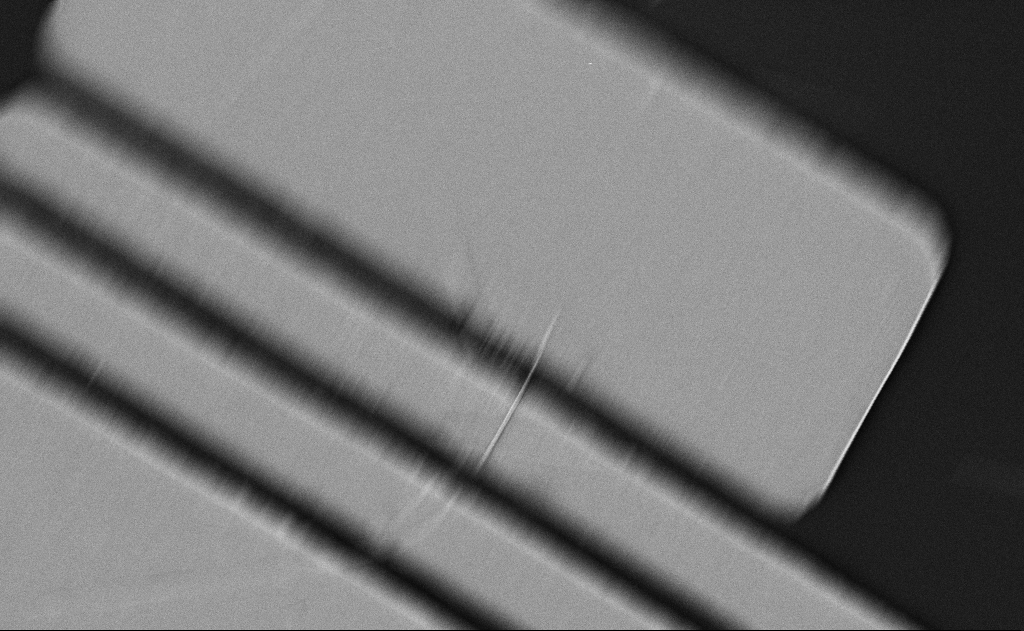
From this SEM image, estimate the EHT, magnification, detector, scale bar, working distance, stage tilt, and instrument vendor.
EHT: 5 kV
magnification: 10.78 K X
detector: SE2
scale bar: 2000 nm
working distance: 21 mm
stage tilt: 0°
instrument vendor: Zeiss